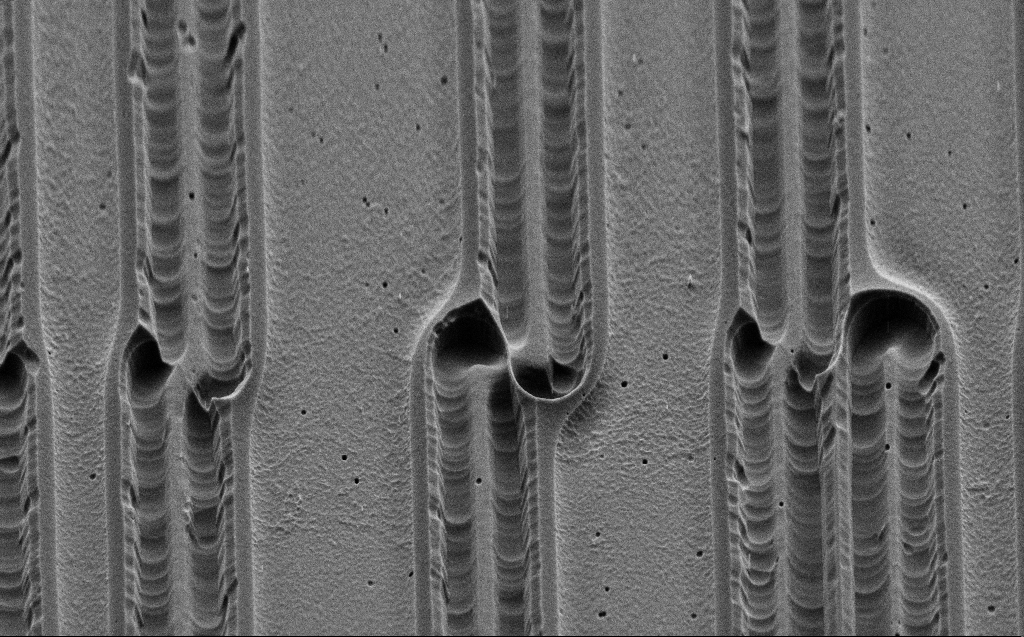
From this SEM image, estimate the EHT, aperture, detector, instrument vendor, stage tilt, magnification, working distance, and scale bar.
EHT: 3 kV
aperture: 30 µm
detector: SE2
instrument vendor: Zeiss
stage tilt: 45°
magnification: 8.42 K X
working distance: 3 mm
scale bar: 2000 nm